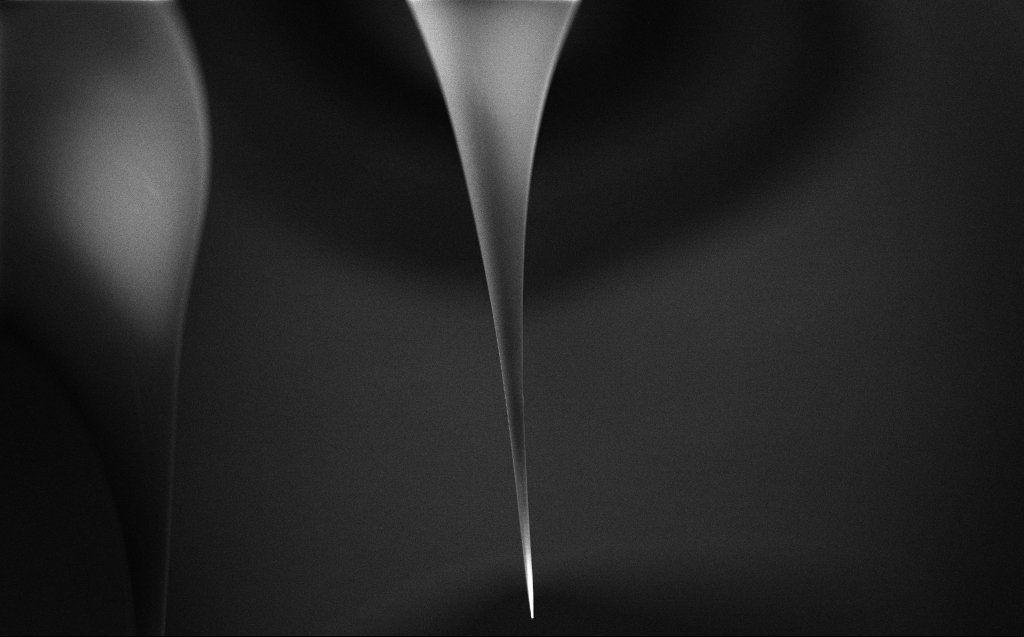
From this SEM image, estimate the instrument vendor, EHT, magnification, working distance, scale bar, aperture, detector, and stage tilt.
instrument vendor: Zeiss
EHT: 2 kV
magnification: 0.1 K X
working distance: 6 mm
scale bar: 200000 nm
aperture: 30 µm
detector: InLens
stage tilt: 45°